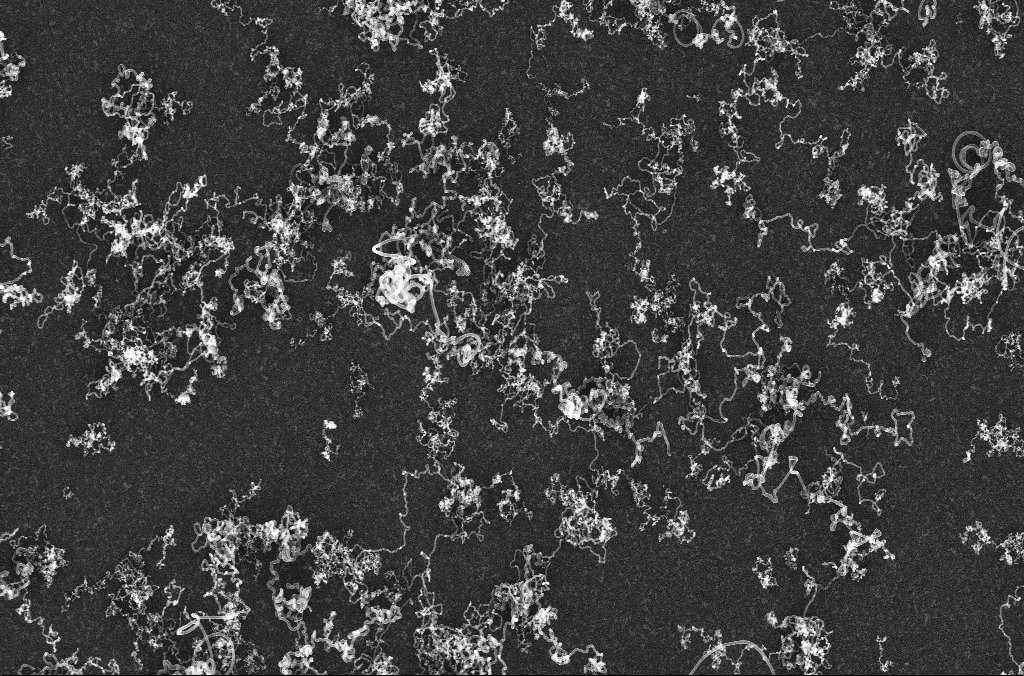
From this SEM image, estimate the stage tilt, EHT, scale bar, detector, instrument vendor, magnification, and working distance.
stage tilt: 0°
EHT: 20 kV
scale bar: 2000 nm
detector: InLens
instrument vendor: Zeiss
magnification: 10 K X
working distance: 4.3 mm